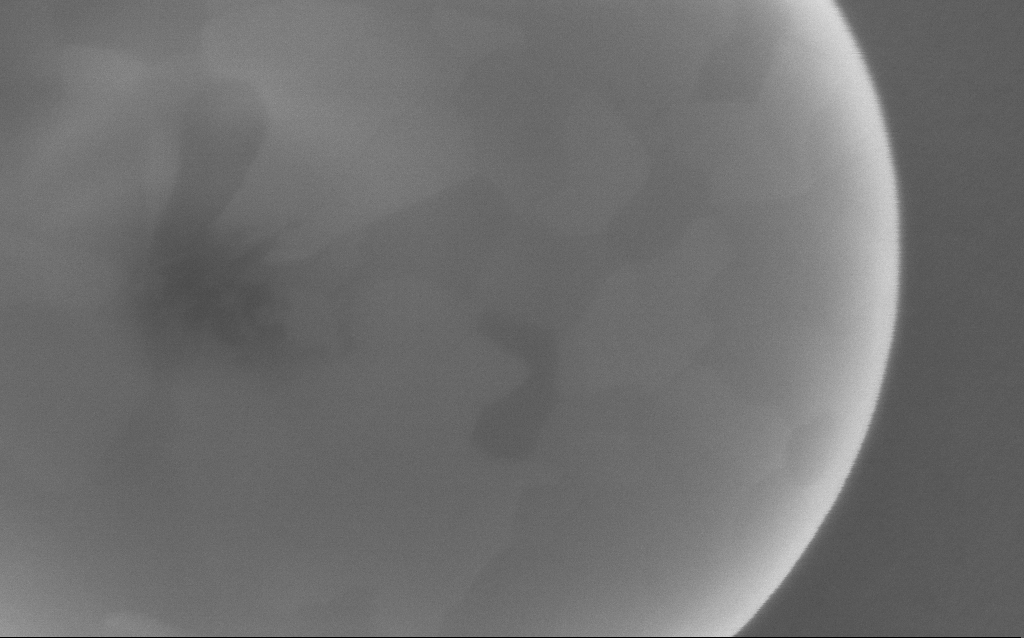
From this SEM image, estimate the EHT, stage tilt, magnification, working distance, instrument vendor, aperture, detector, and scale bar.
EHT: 5 kV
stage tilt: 0°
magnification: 254.35 K X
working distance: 2 mm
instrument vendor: Zeiss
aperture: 30 µm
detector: InLens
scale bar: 200 nm